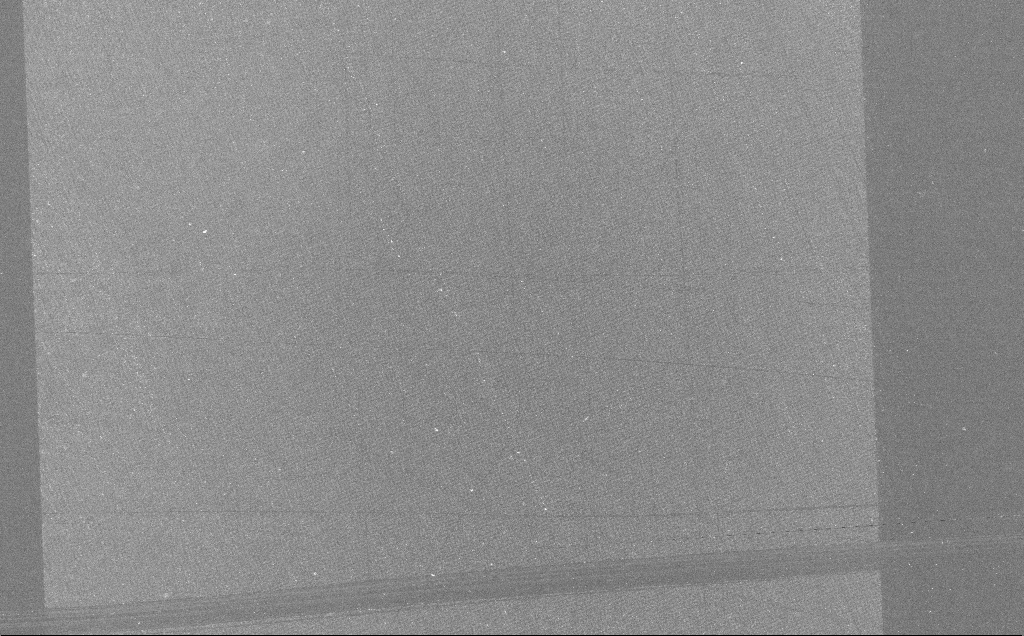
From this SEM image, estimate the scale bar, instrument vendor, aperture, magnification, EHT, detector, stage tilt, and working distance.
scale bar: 100000 nm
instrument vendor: Zeiss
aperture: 30 µm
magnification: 0.41 K X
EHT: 10 kV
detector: InLens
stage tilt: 0°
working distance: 7 mm